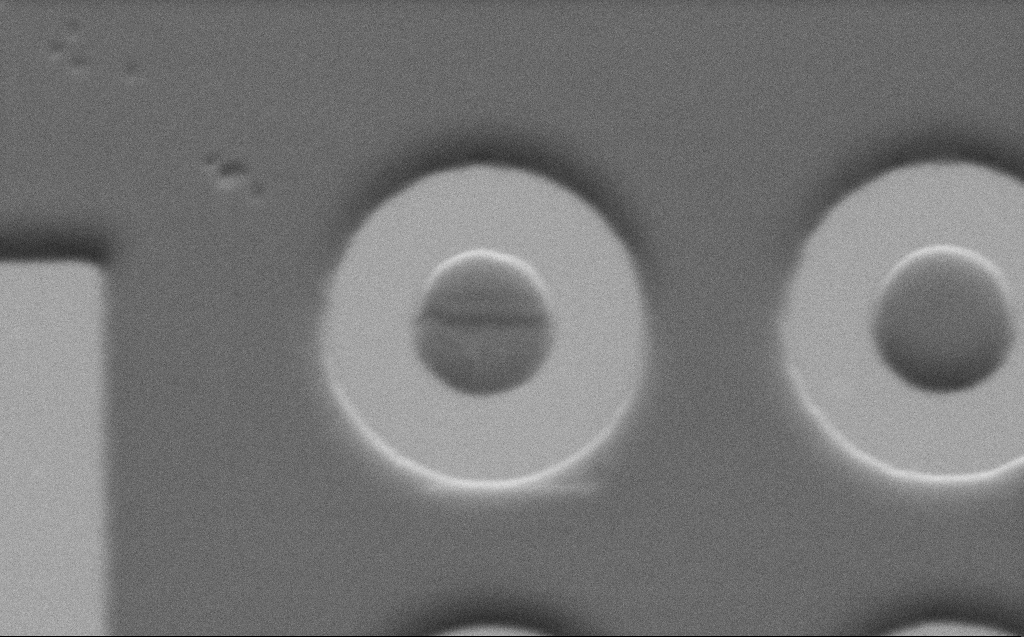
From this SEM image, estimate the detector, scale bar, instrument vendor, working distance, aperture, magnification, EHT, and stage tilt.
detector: SE2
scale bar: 1000 nm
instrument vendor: Zeiss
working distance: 6 mm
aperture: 30 µm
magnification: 16.87 K X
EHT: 3 kV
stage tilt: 45°